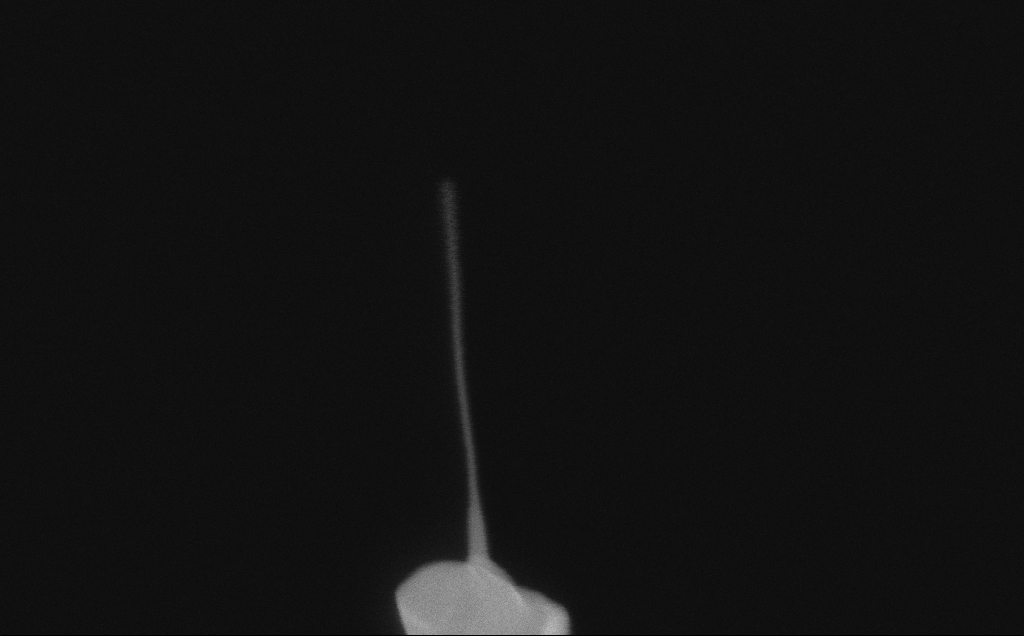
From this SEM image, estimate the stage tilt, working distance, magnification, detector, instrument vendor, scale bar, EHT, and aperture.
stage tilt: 0°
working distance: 6 mm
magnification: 257.27 K X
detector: InLens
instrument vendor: Zeiss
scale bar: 200 nm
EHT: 10 kV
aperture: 30 µm